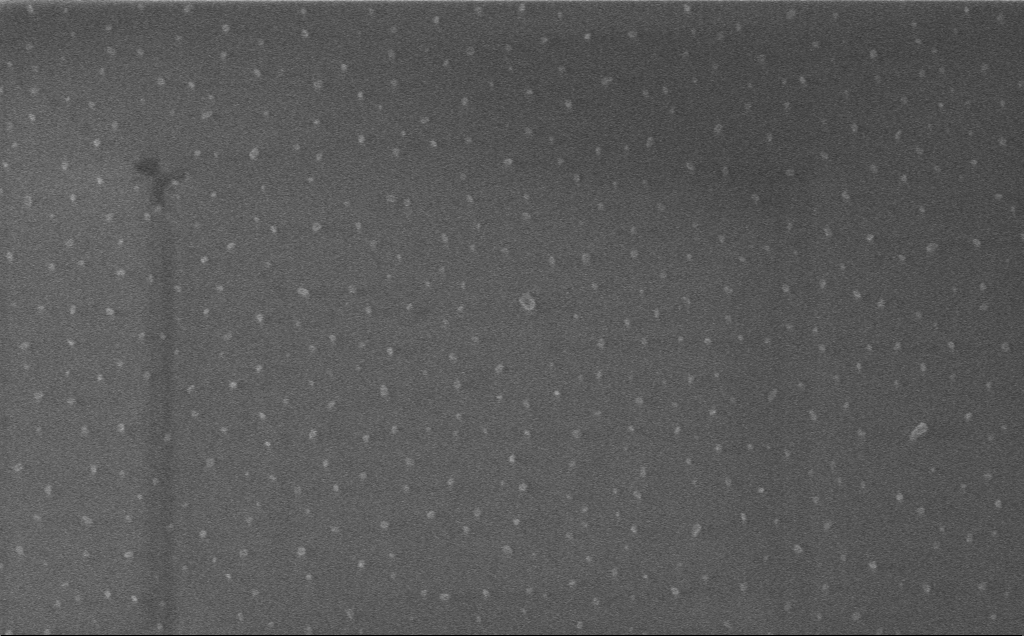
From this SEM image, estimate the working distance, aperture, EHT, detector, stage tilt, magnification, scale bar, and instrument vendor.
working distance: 3 mm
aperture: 30 µm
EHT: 1 kV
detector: InLens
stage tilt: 0°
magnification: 35.66 K X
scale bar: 1000 nm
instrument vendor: Zeiss